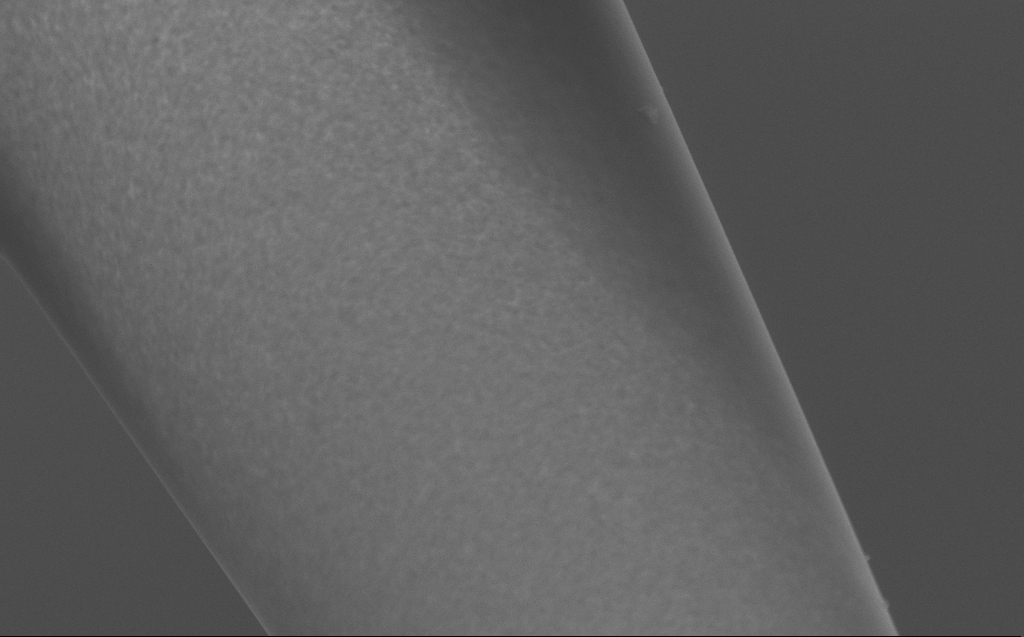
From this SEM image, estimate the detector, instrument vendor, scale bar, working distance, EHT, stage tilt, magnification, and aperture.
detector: SE2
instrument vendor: Zeiss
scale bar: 2000 nm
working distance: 4 mm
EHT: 5 kV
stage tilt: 45°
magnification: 31.69 K X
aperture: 30 µm